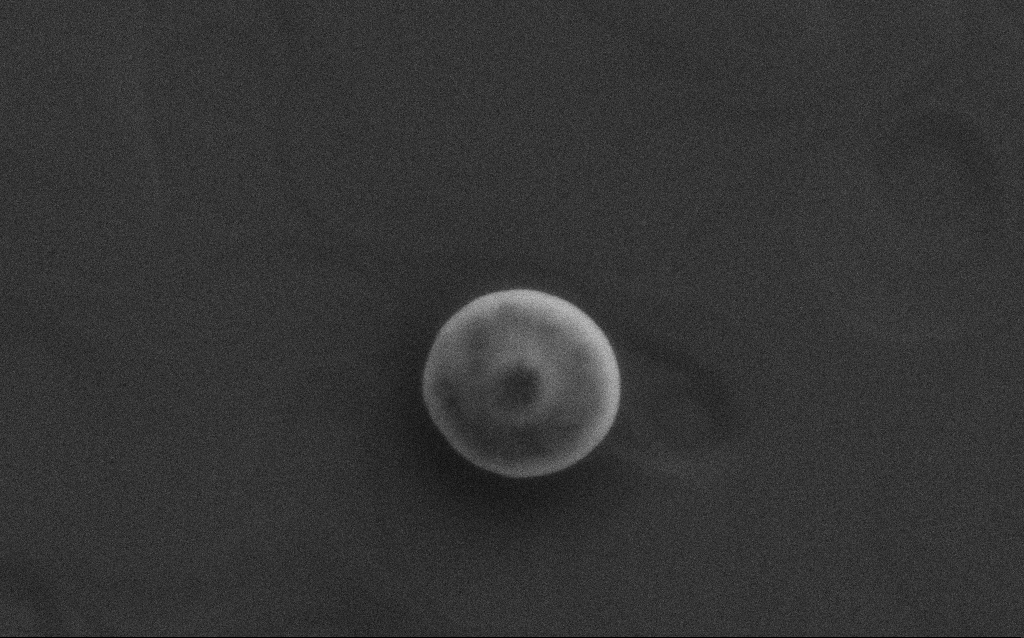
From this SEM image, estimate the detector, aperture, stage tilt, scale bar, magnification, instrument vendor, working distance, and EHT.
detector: SE2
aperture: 30 µm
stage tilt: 0°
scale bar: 1000 nm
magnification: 58.16 K X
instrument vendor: Zeiss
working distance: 2 mm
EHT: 10 kV